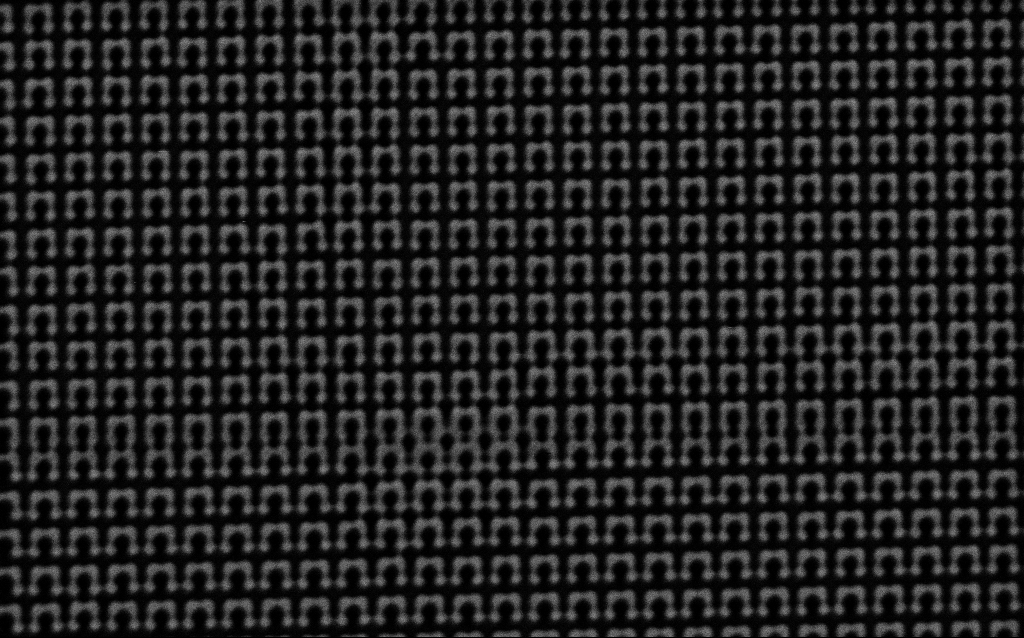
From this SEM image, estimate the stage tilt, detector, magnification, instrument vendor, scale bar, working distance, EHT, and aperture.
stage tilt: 0°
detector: SE2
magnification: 30.15 K X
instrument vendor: Zeiss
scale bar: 2000 nm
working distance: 8 mm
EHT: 1.5 kV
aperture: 30 µm